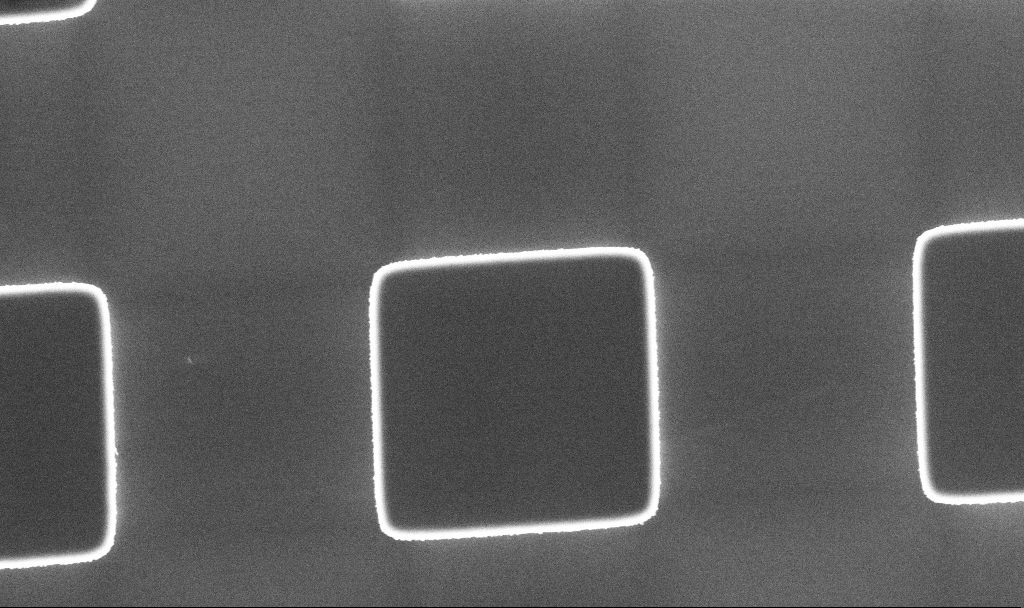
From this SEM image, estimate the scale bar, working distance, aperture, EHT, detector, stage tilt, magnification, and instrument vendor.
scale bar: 2000 nm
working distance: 5.3 mm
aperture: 30 µm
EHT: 5 kV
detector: InLens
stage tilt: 0°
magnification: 12.55 K X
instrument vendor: Zeiss